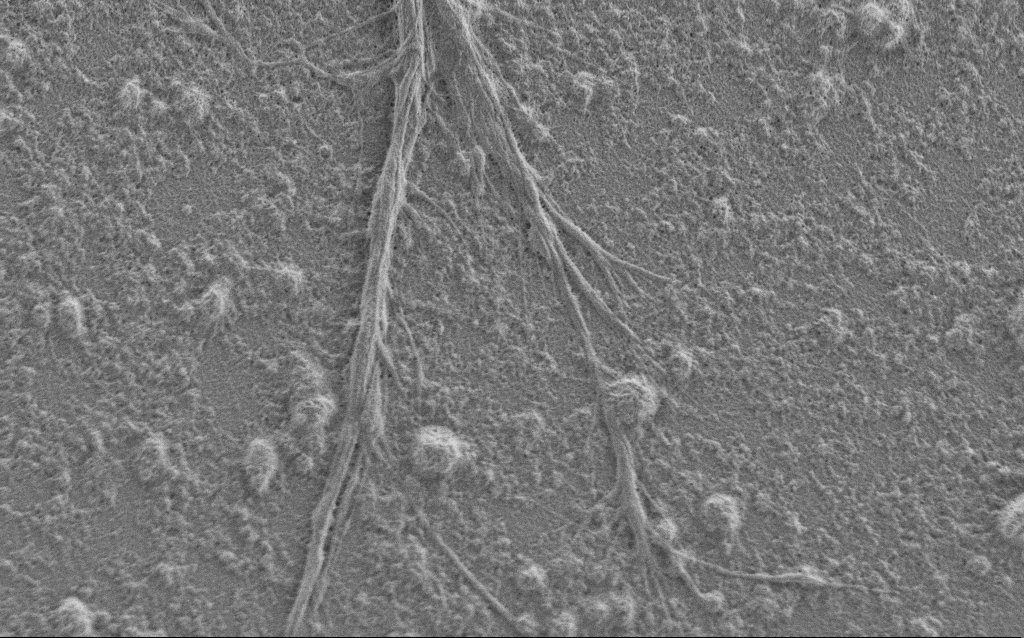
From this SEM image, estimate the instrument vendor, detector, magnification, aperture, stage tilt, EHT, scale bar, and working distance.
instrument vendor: Zeiss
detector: SE2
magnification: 7.5 K X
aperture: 30 µm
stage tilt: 0°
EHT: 1 kV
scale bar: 2000 nm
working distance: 6 mm